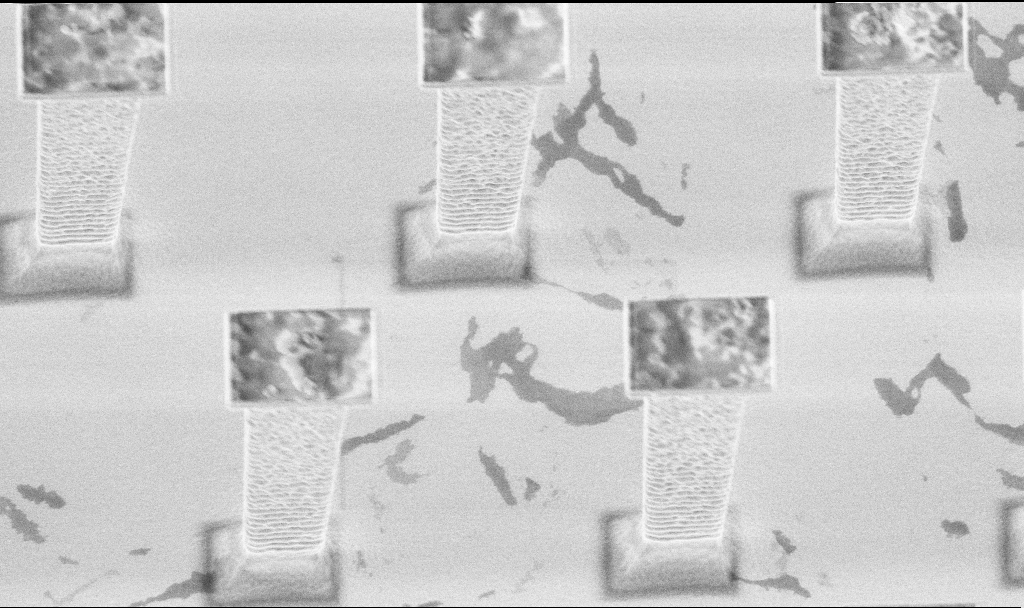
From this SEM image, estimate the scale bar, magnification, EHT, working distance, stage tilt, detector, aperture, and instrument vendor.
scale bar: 2000 nm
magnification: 12.16 K X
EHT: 3 kV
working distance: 5.7 mm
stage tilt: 20°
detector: InLens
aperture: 30 µm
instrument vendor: Zeiss